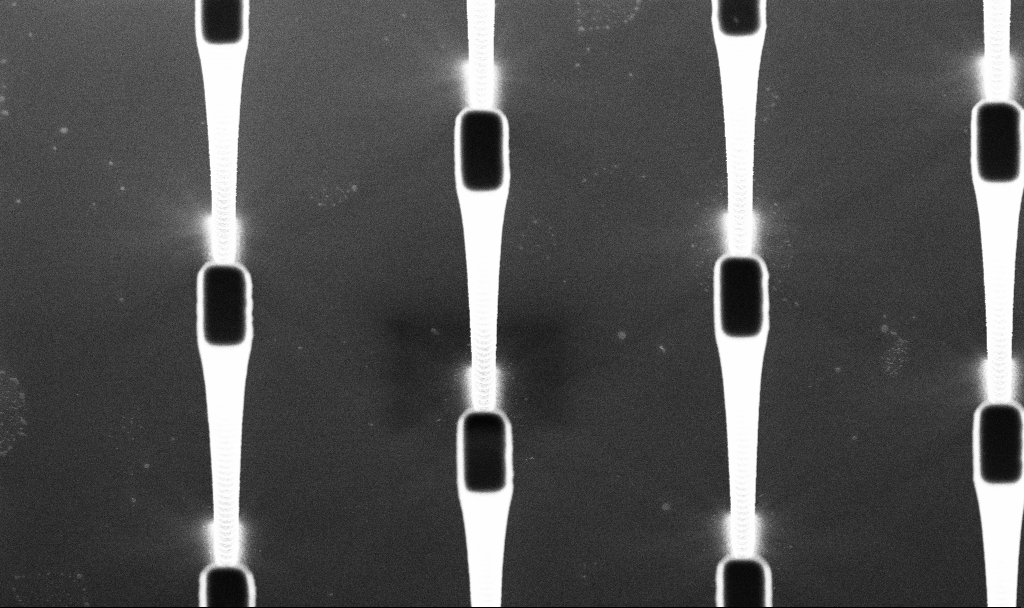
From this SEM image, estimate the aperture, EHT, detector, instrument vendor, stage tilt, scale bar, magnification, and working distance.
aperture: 30 µm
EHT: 5 kV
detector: InLens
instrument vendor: Zeiss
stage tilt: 29.2°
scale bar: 2000 nm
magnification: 10.56 K X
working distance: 6.9 mm